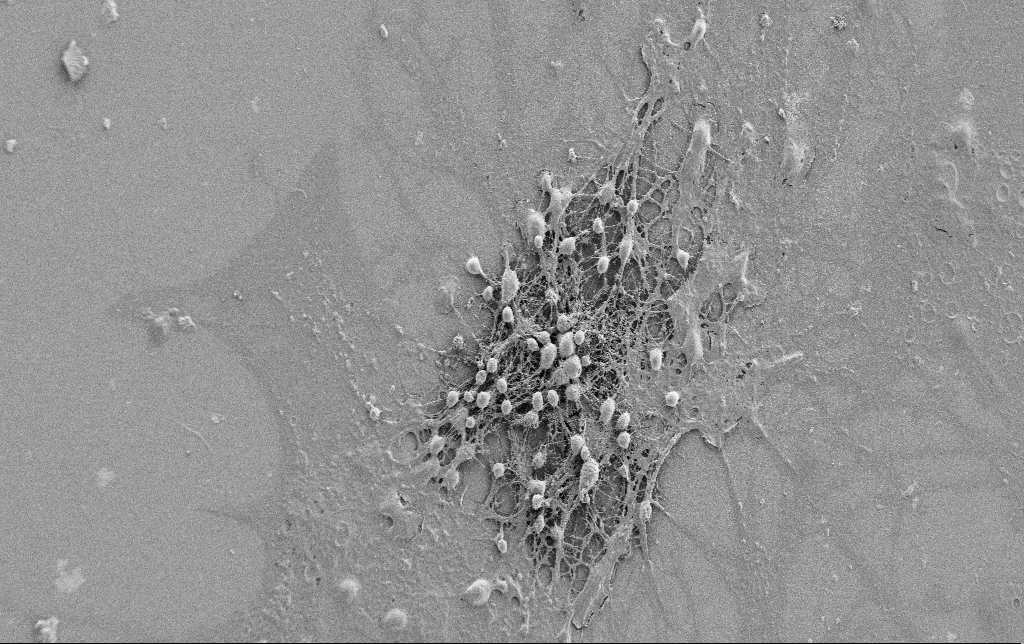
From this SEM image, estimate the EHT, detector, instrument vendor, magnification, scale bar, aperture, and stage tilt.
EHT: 0.9 kV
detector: SE2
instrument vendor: Zeiss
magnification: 1 K X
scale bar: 20000 nm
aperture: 30 µm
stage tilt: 0°